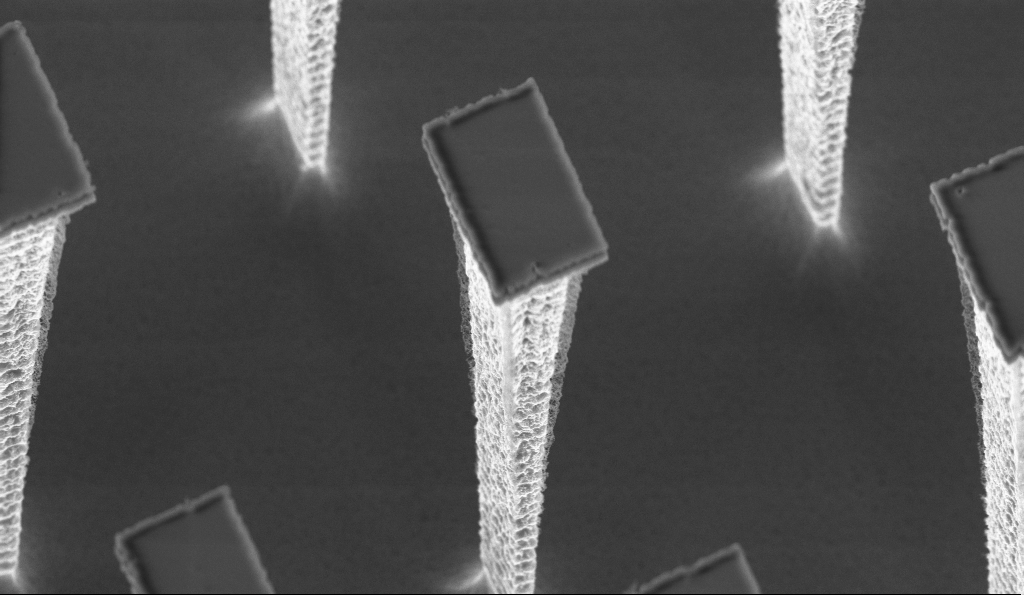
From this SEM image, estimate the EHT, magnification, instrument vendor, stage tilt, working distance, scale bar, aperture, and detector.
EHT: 5 kV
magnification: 15.7 K X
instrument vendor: Zeiss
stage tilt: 30°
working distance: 5.5 mm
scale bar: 2000 nm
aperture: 30 µm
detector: InLens